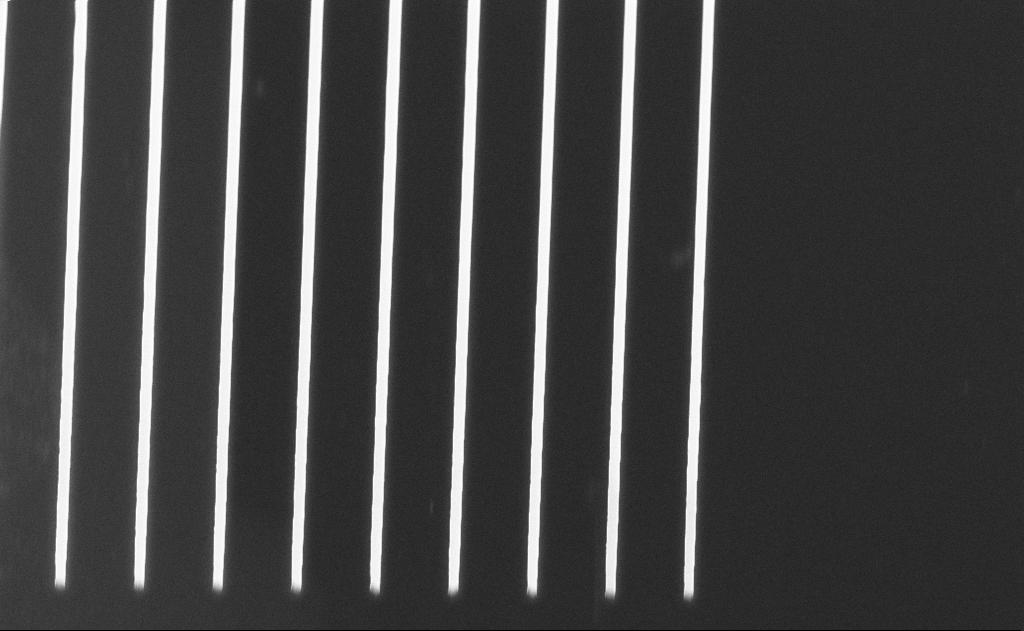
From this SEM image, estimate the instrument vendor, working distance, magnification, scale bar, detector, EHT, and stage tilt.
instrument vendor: Zeiss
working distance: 18 mm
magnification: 3.61 K X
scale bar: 20000 nm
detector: SE2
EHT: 10 kV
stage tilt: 50°